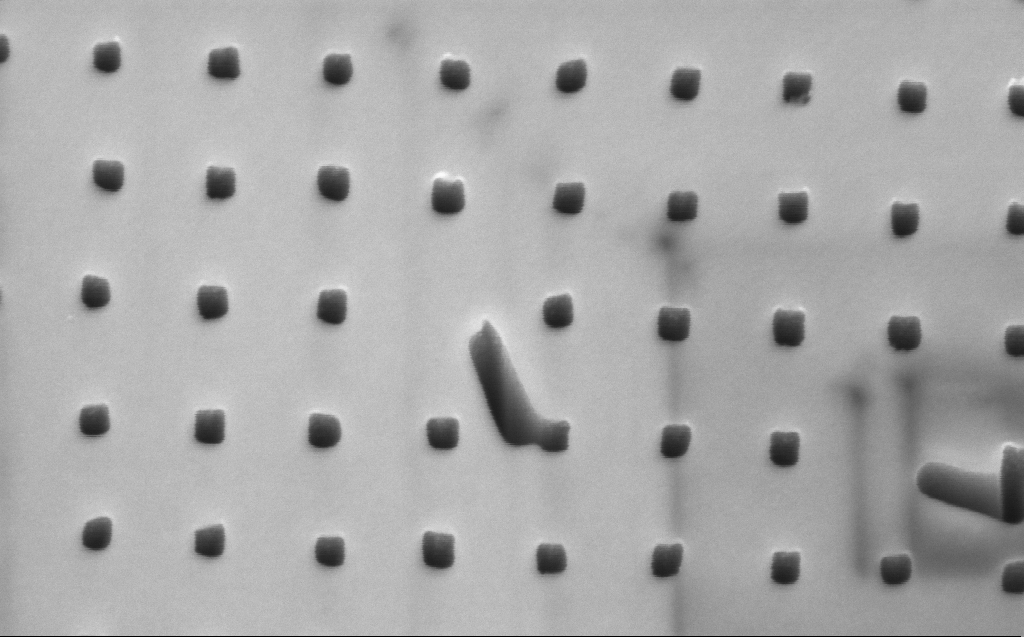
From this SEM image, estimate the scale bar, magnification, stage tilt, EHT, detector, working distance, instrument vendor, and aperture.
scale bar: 200 nm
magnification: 85.14 K X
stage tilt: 45°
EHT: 3 kV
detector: InLens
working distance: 6 mm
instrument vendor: Zeiss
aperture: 30 µm